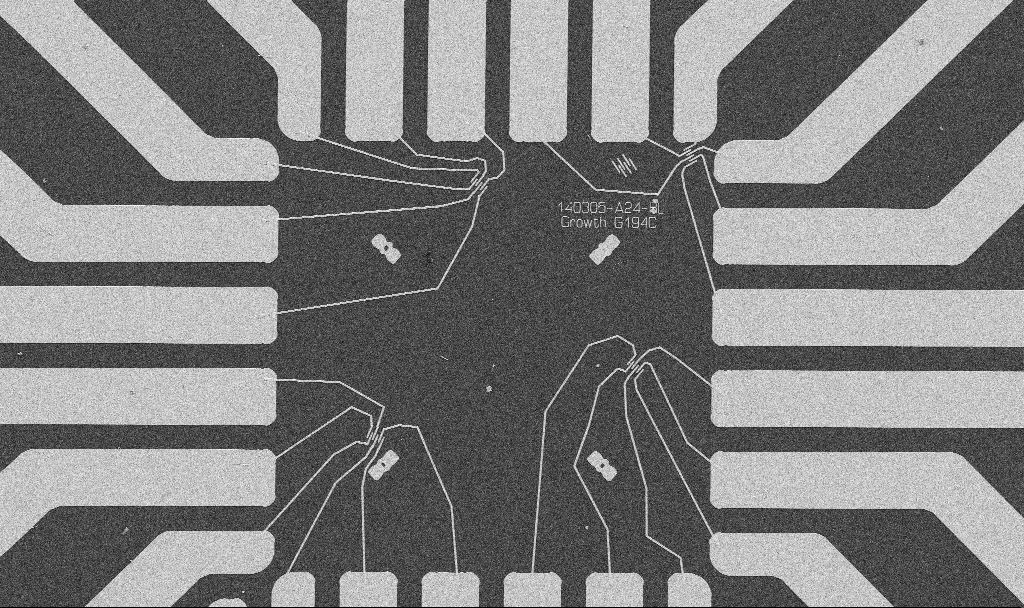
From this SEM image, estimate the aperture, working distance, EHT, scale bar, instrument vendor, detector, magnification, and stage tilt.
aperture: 30 µm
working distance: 10.7 mm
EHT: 5 kV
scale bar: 20000 nm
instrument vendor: Zeiss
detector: SE2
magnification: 1 K X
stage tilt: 0°